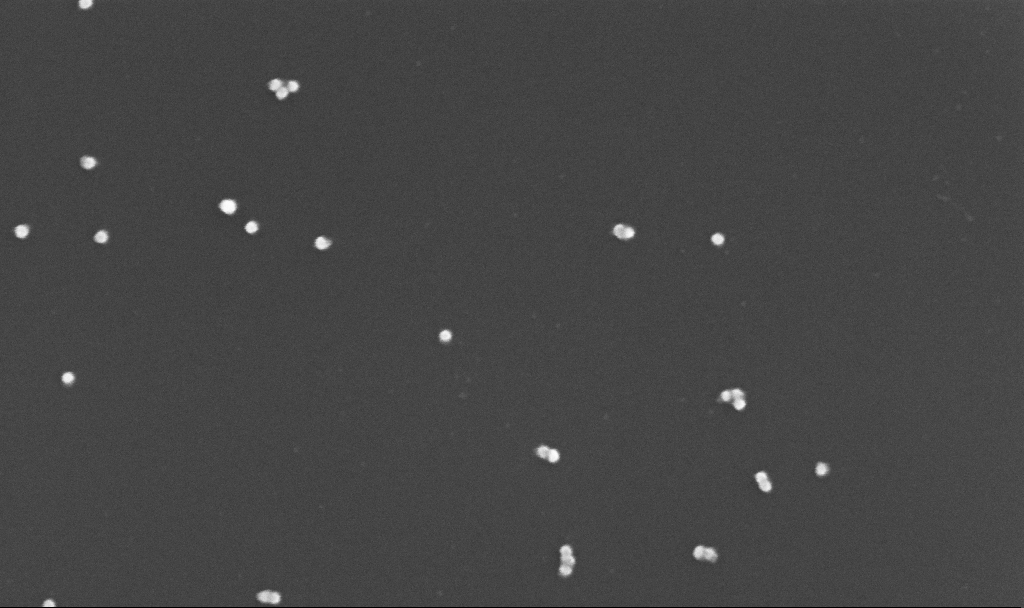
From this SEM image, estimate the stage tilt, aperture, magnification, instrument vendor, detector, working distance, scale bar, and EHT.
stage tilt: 0°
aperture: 30 µm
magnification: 200 K X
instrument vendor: Zeiss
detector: InLens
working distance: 3.4 mm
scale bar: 100 nm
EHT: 10 kV